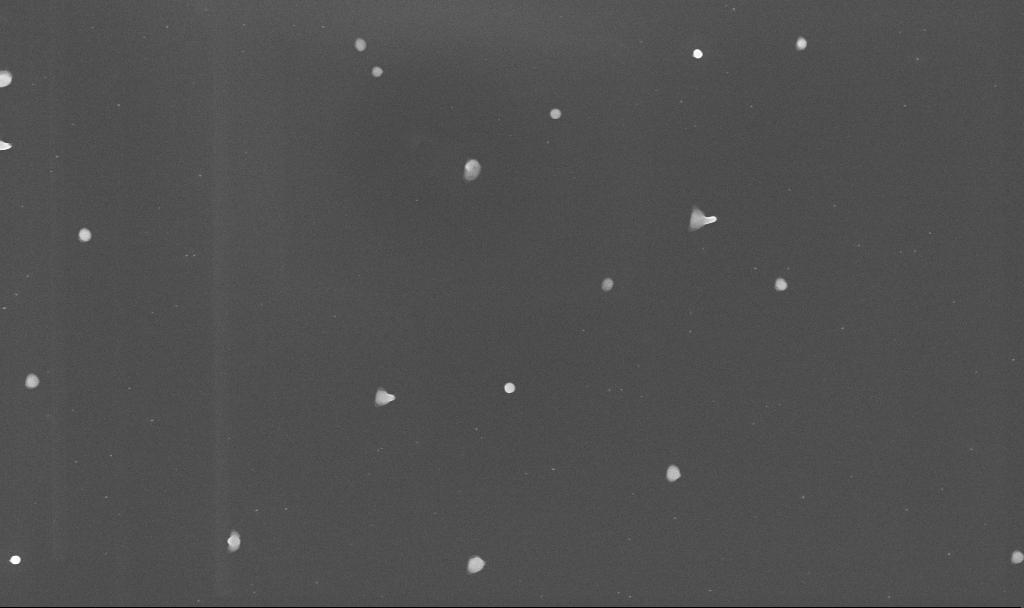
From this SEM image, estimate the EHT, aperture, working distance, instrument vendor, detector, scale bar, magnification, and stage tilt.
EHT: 10 kV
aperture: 30 µm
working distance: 3.4 mm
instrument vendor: Zeiss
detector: InLens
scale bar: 1000 nm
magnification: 50.55 K X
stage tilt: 0°